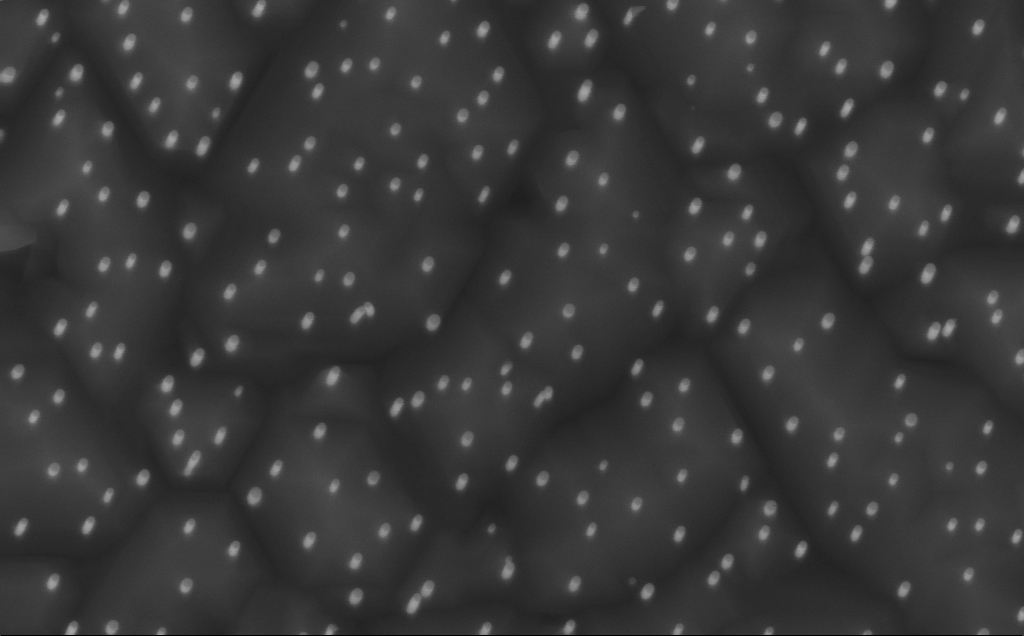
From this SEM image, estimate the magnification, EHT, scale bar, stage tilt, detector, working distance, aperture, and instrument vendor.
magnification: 80 K X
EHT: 10 kV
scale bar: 200 nm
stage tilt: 0°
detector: InLens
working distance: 5 mm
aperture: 30 µm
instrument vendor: Zeiss